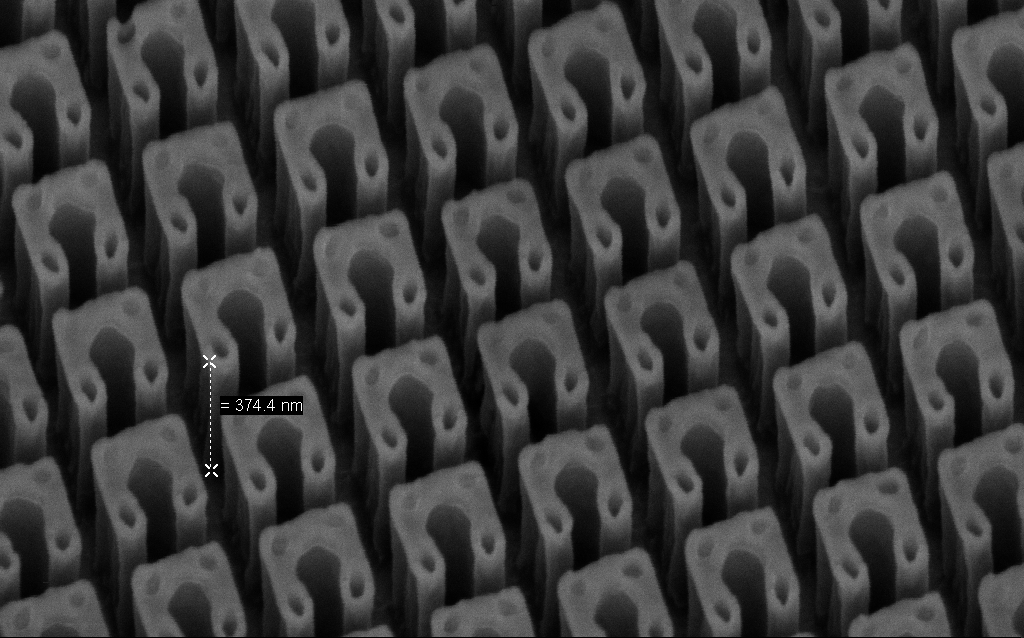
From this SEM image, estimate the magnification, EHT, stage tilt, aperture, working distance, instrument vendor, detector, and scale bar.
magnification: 106.91 K X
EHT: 3 kV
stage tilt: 45°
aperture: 30 µm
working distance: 7.3 mm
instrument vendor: Zeiss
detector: InLens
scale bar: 200 nm